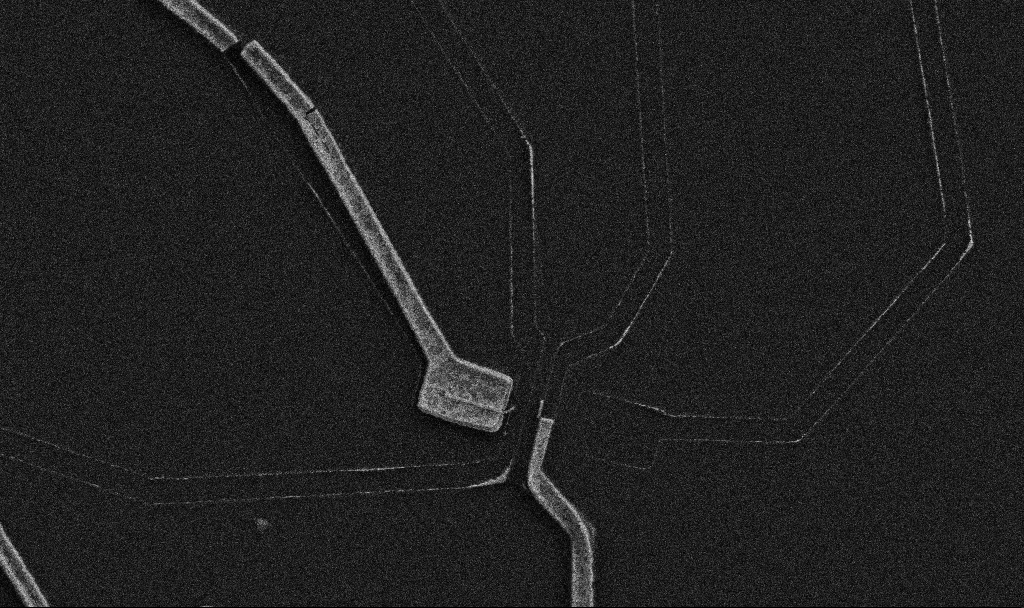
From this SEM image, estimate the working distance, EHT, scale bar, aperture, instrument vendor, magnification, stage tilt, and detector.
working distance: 9.7 mm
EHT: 5 kV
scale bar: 2000 nm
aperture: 30 µm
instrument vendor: Zeiss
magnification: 10 K X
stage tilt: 0°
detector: SE2